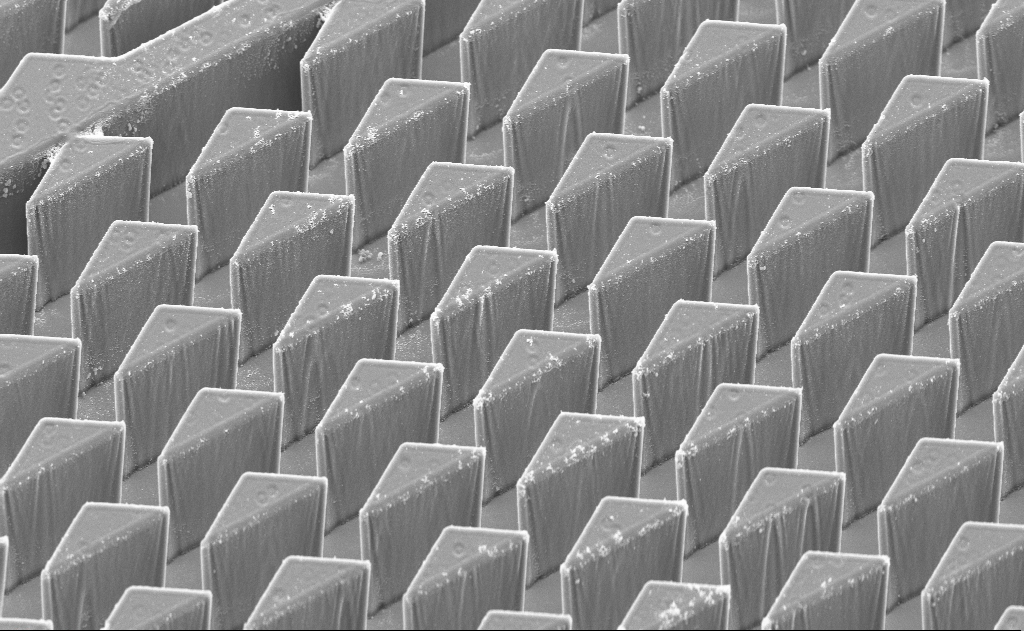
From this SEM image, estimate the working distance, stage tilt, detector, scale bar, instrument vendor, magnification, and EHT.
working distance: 11 mm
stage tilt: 45°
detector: SE2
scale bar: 20000 nm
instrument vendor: Zeiss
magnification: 1.45 K X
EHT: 10 kV